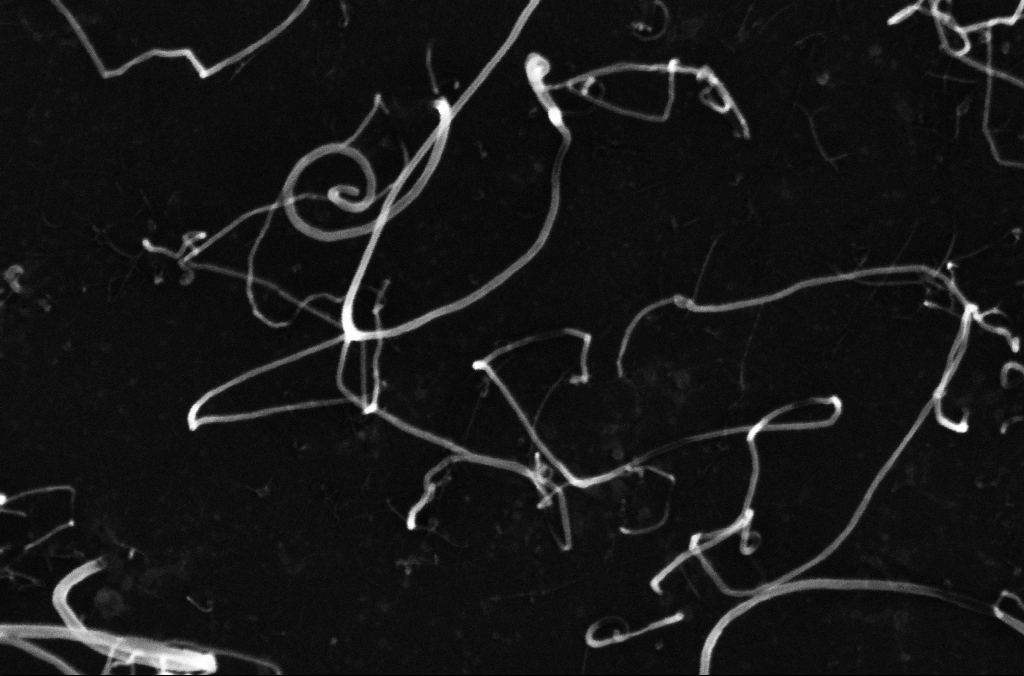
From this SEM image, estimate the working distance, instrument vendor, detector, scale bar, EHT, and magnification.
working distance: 3.3 mm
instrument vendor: Zeiss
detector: InLens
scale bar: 100 nm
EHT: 10 kV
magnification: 200 K X